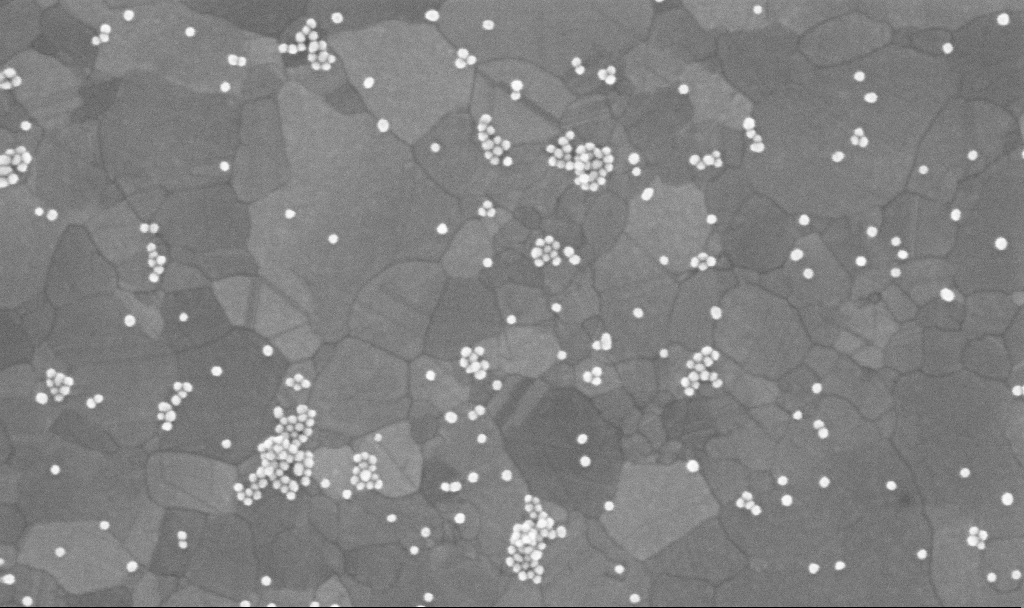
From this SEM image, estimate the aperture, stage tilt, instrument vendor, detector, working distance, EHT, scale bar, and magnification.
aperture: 30 µm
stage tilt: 0°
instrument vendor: Zeiss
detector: InLens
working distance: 3.8 mm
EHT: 10 kV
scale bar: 100 nm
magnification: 200 K X